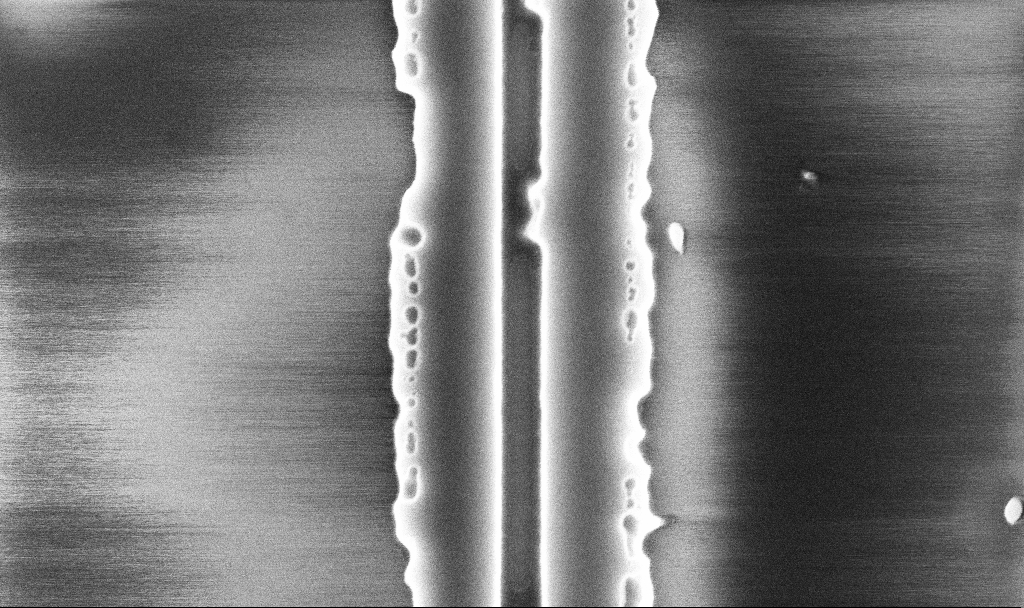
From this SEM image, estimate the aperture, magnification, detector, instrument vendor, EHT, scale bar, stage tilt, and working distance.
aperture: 30 µm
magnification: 64.63 K X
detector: InLens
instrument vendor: Zeiss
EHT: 5 kV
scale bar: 1000 nm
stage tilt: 0°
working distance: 10.1 mm